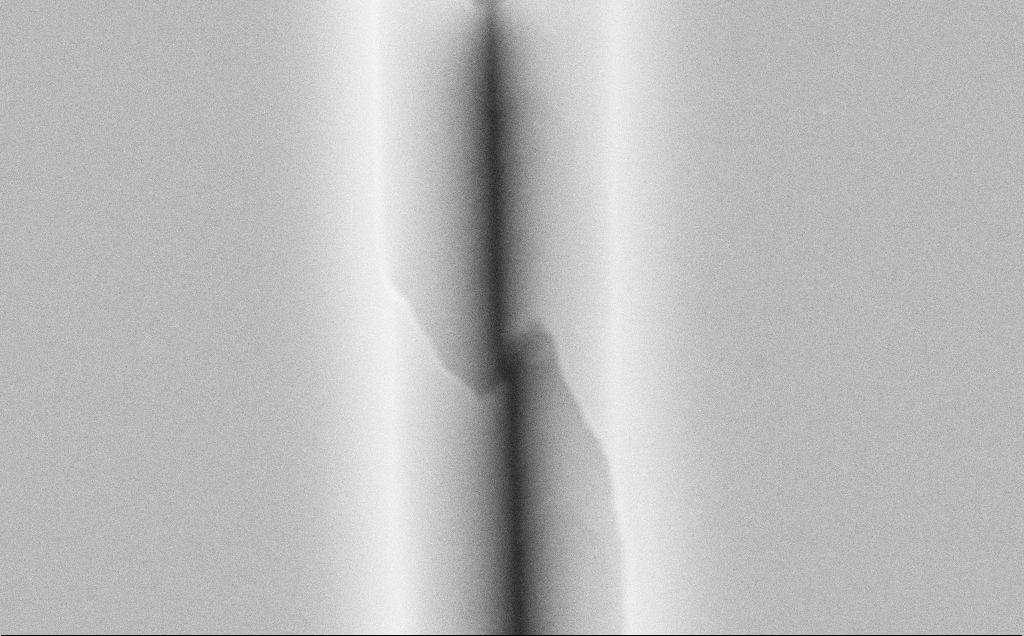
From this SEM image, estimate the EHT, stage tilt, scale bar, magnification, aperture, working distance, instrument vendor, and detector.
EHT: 10 kV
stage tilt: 0°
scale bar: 1000 nm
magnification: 47.83 K X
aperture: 30 µm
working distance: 10 mm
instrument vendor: Zeiss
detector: SE2